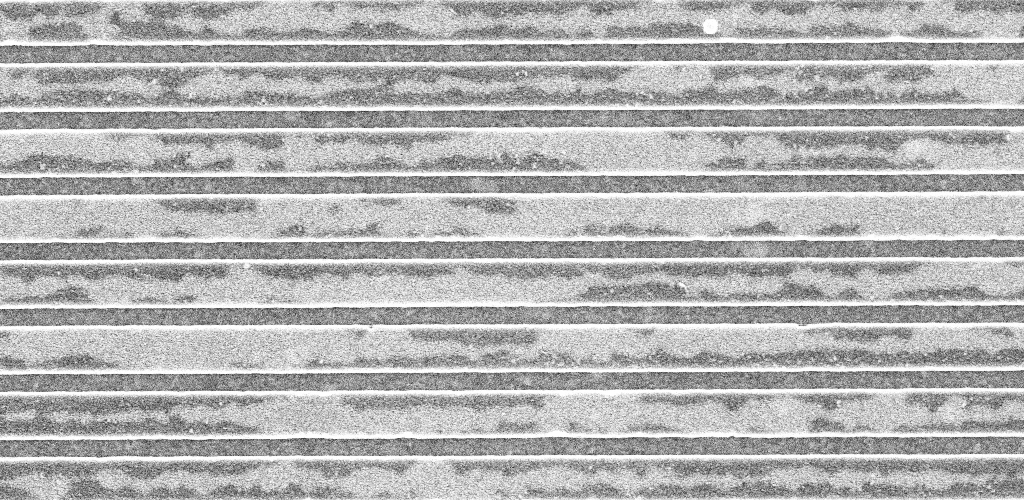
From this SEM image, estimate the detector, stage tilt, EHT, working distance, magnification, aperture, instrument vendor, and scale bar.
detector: InLens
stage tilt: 0°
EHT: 5 kV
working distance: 3.1 mm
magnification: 40.48 K X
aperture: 30 µm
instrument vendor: Zeiss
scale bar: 1000 nm